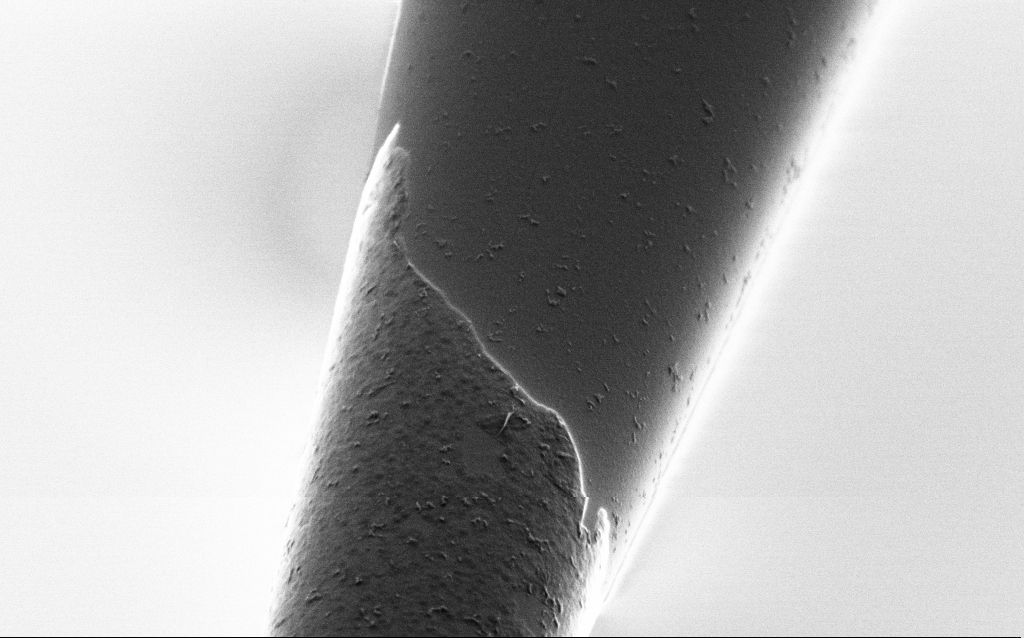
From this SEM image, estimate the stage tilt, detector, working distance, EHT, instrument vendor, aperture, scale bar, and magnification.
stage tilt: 45°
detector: SE2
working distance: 6 mm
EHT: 1 kV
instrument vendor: Zeiss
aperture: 30 µm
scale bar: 2000 nm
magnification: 25 K X